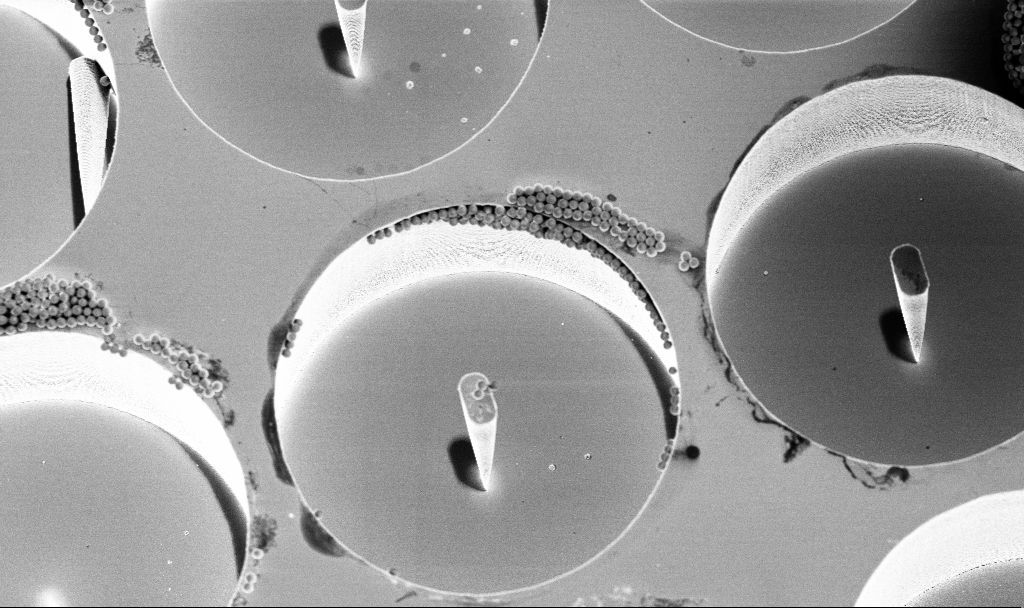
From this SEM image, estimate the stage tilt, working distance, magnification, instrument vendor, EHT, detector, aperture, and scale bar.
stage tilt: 15°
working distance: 4.7 mm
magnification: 5.03 K X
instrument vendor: Zeiss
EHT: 4 kV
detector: InLens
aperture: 30 µm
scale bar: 10000 nm